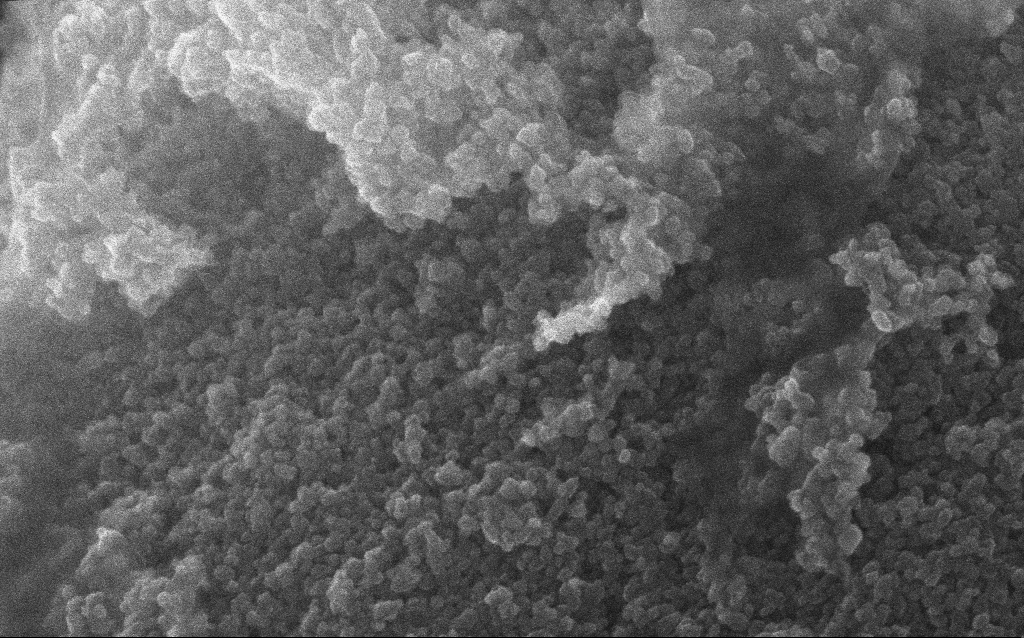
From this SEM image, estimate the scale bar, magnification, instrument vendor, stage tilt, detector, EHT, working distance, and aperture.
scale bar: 100 nm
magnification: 211.33 K X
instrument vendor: Zeiss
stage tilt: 0°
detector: InLens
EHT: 10 kV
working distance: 2.8 mm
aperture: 30 µm